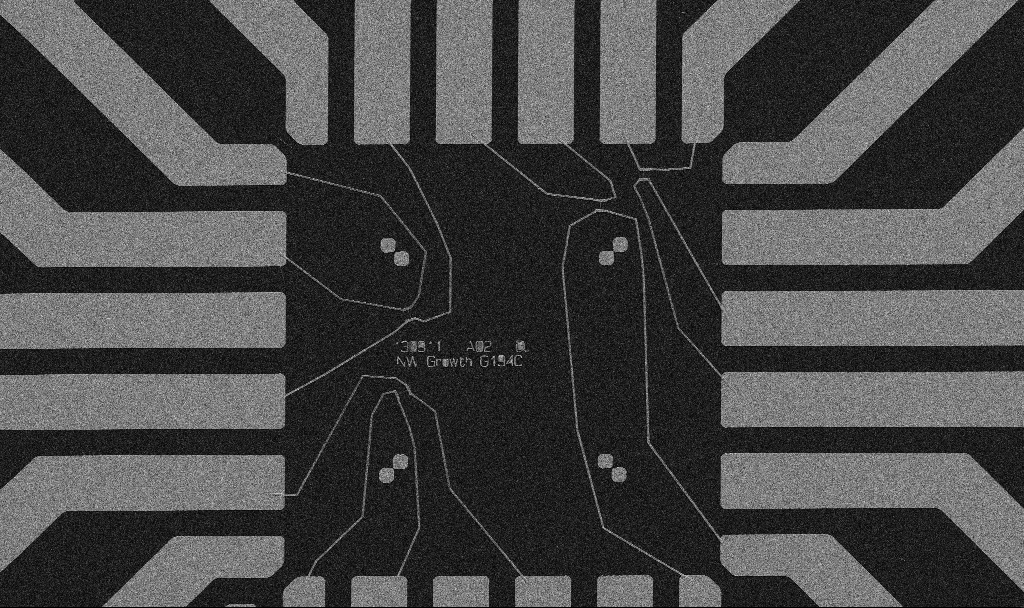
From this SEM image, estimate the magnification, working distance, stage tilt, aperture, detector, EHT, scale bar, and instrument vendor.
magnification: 1 K X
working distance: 10.7 mm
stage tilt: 0°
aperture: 30 µm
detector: SE2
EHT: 5 kV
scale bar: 20000 nm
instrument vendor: Zeiss